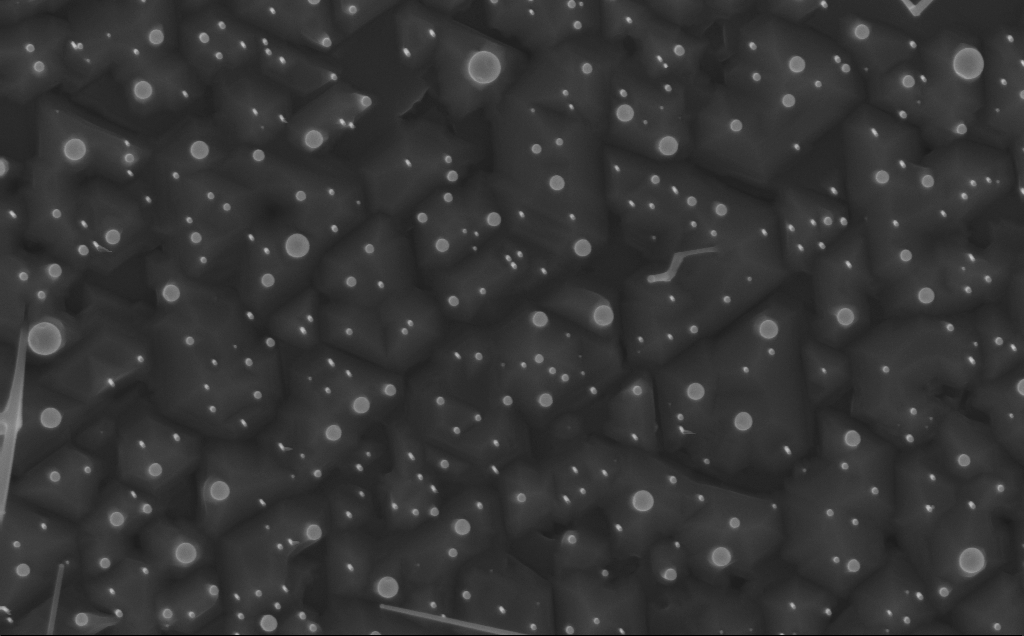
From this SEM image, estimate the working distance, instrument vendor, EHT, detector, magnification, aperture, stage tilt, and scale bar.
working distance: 3 mm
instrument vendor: Zeiss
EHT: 10 kV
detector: InLens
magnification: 40 K X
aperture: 30 µm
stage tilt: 0°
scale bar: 1000 nm